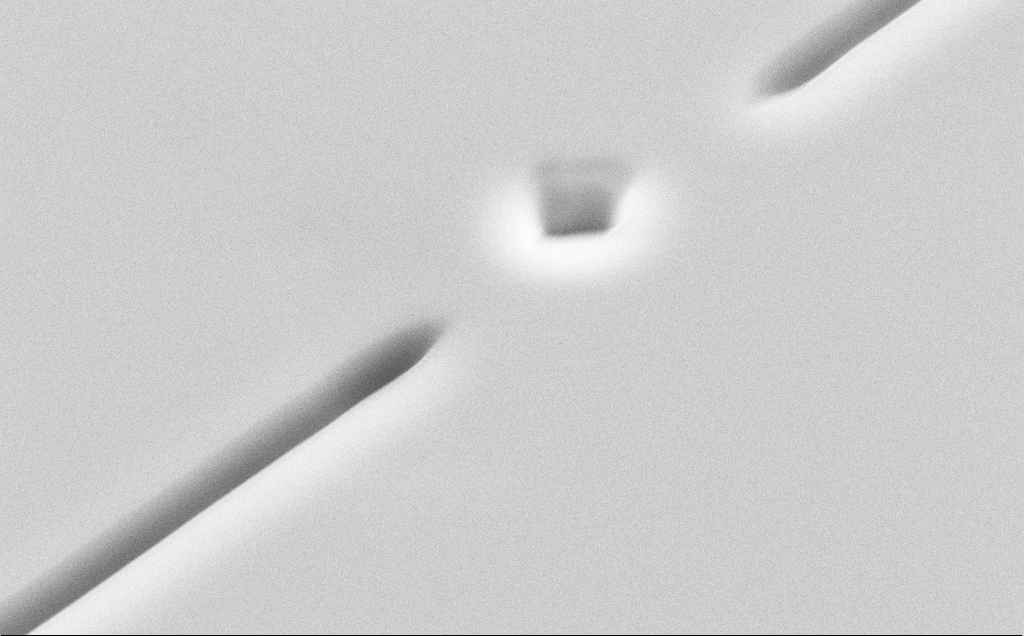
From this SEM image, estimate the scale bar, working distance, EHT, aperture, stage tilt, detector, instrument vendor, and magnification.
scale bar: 2000 nm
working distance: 13 mm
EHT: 10 kV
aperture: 30 µm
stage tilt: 45°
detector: SE2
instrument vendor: Zeiss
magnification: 17.76 K X